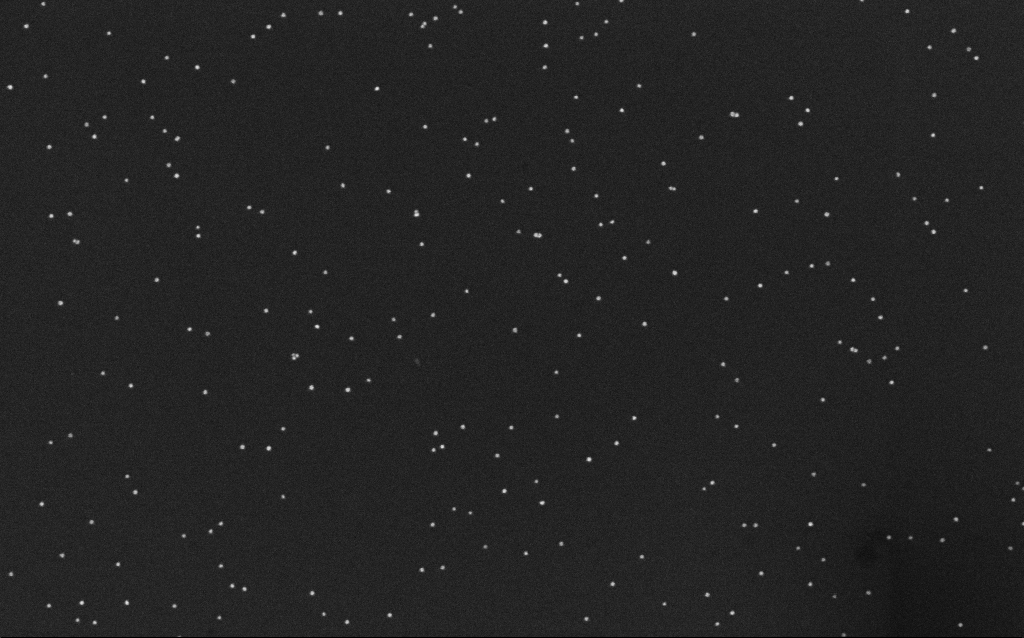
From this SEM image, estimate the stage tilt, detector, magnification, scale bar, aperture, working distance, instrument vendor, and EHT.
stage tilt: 0°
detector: InLens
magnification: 100 K X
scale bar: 200 nm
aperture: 30 µm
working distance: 6.5 mm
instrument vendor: Zeiss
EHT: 10 kV